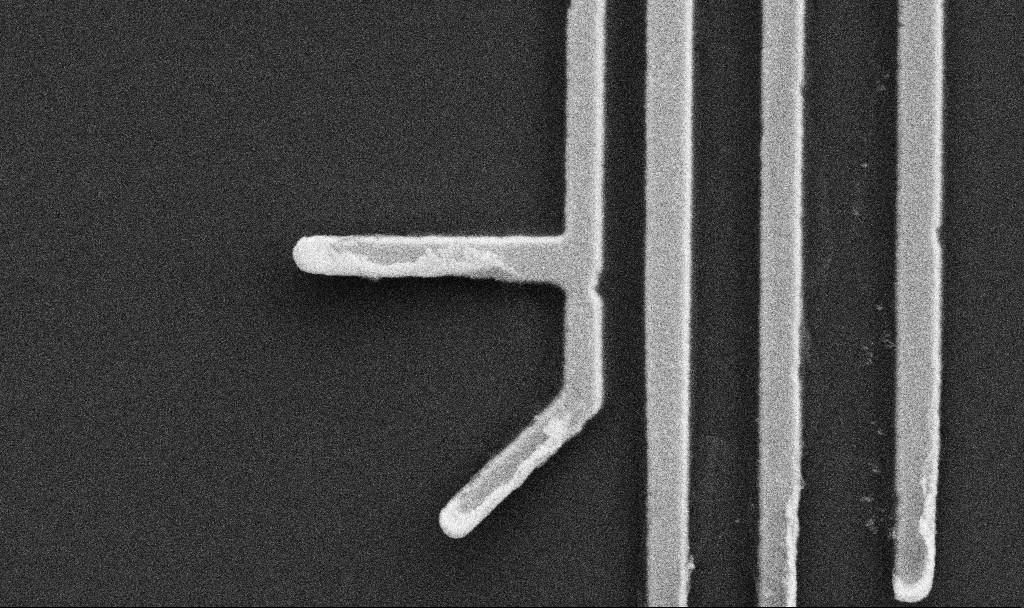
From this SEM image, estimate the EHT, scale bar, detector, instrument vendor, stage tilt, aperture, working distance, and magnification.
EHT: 5 kV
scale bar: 1000 nm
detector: SE2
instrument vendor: Zeiss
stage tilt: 0°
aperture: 30 µm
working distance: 10.7 mm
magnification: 50.21 K X